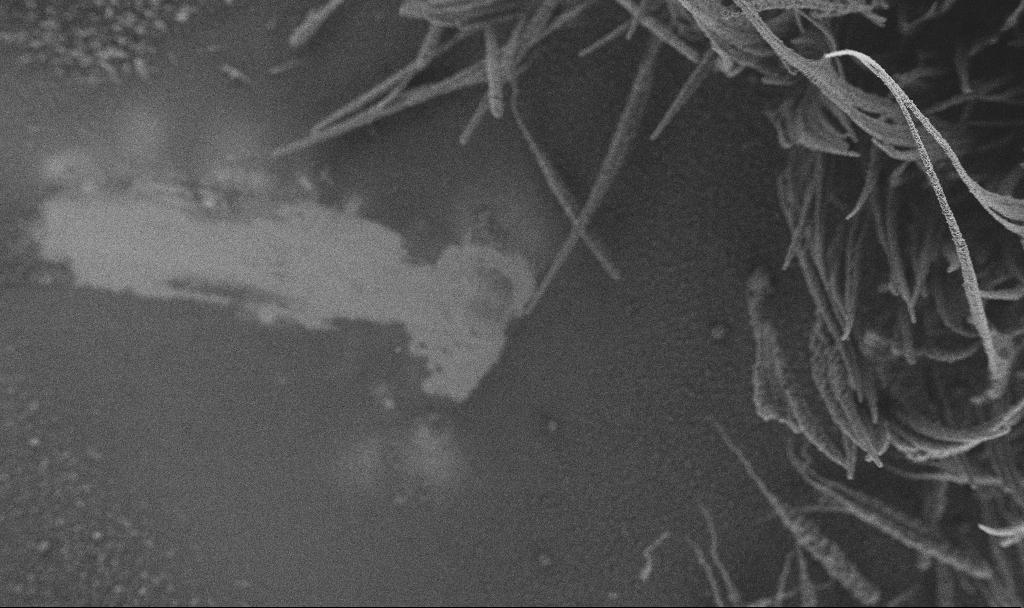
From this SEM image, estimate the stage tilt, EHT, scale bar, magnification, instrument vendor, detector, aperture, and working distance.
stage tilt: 0°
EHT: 3 kV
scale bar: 100000 nm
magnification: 0.15 K X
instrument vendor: Zeiss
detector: SE2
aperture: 30 µm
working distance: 2.9 mm